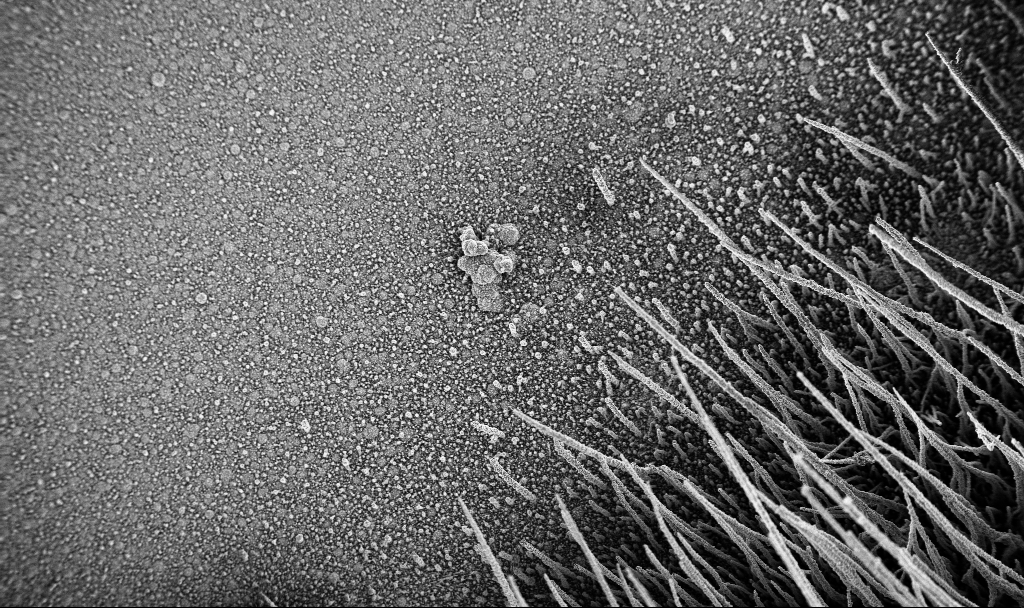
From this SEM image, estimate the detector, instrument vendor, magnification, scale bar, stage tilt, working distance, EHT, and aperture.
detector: InLens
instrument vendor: Zeiss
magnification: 0.15 K X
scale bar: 100000 nm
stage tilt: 0°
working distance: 3.8 mm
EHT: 3 kV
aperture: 30 µm